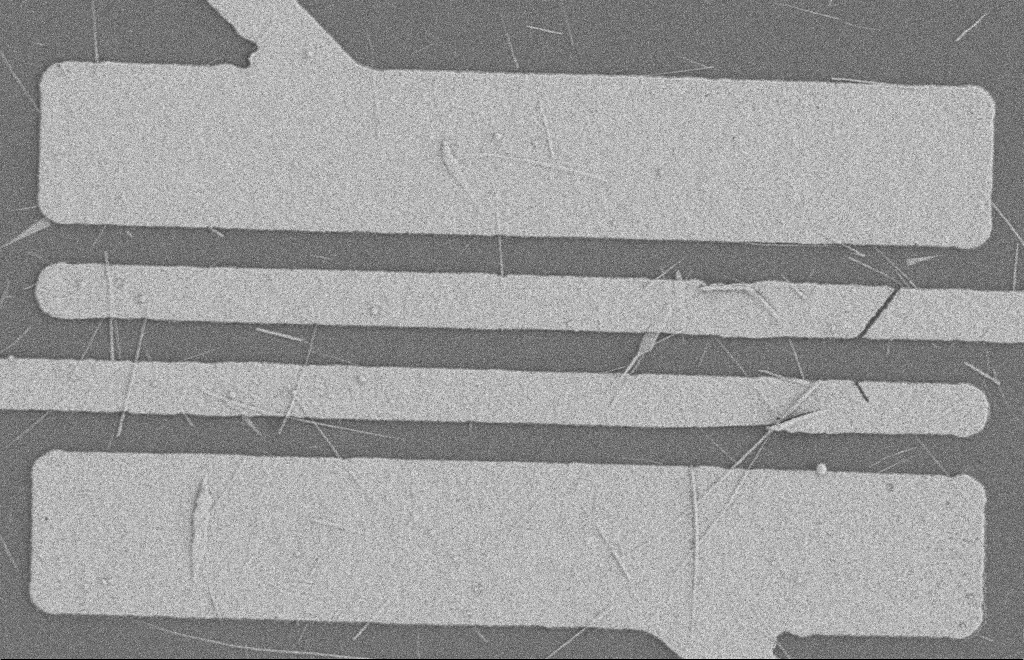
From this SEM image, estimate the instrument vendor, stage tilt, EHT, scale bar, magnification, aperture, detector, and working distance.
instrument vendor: Zeiss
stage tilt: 0°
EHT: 2 kV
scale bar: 2000 nm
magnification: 5.73 K X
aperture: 20 µm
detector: SE2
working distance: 8 mm